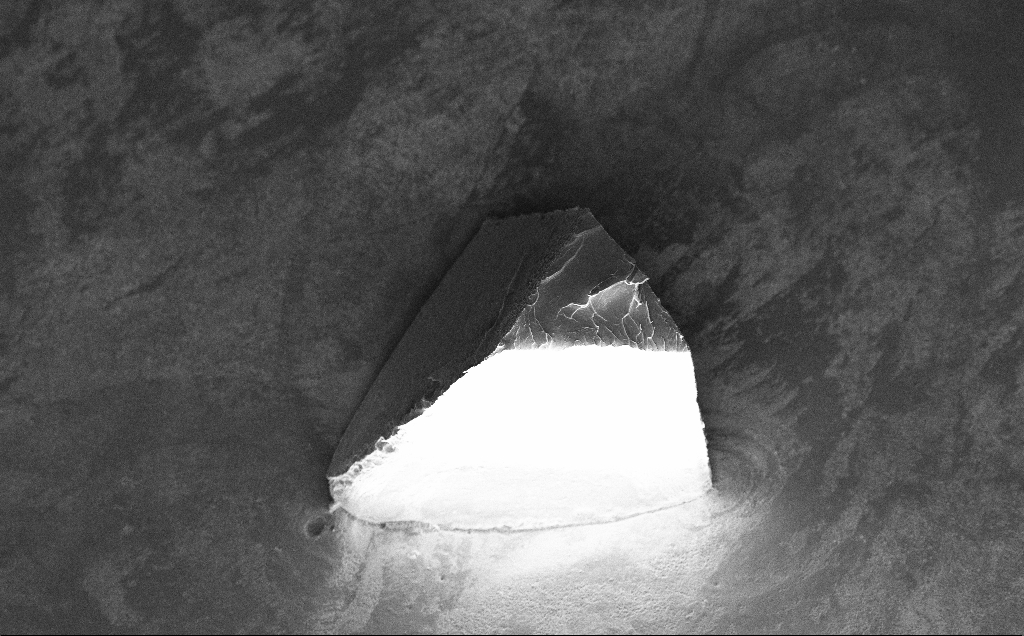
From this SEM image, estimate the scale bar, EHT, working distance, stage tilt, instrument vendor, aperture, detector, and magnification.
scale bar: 100000 nm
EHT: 10 kV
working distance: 9 mm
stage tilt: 30°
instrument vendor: Zeiss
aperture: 30 µm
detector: InLens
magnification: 0.238 K X